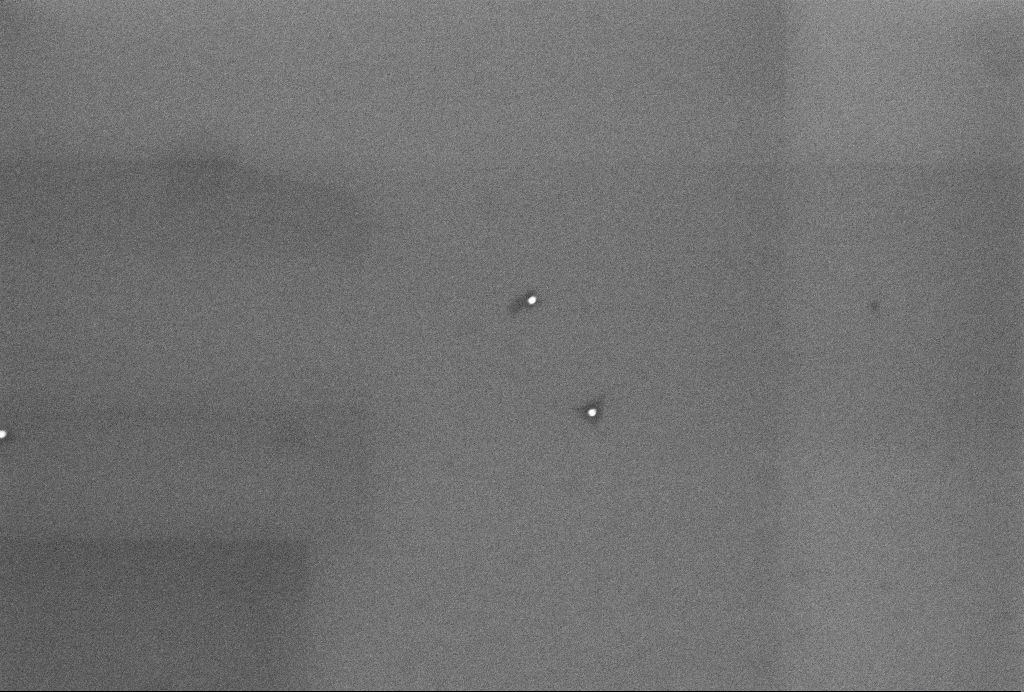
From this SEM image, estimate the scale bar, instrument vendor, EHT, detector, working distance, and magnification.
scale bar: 200 nm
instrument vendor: Zeiss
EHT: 4 kV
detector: InLens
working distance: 3.2 mm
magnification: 100 K X